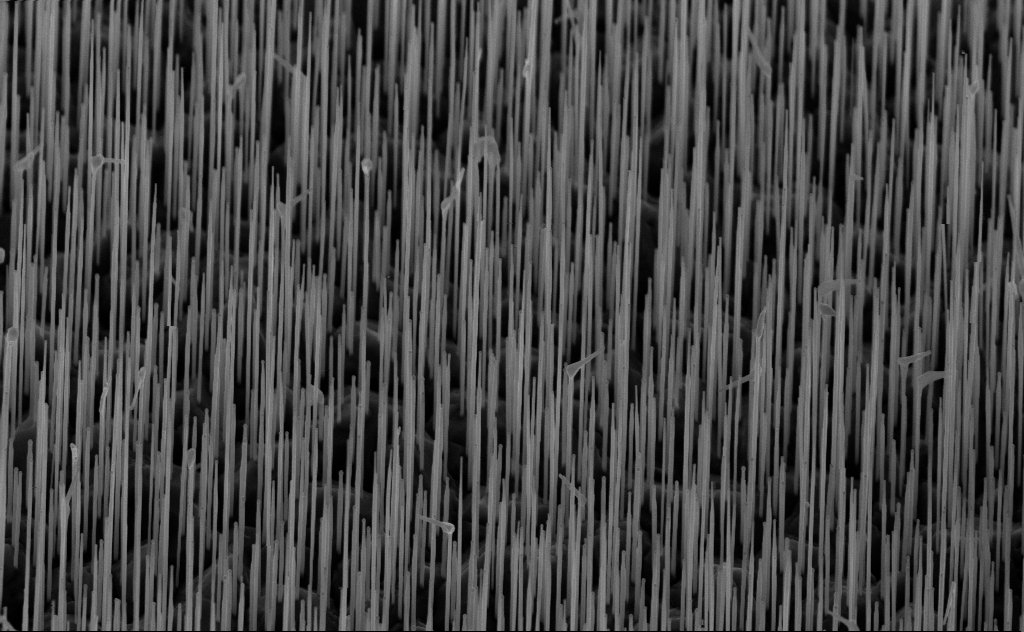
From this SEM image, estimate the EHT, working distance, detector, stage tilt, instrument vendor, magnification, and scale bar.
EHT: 10 kV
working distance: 6 mm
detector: InLens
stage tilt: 45°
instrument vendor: Zeiss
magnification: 20 K X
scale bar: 1000 nm